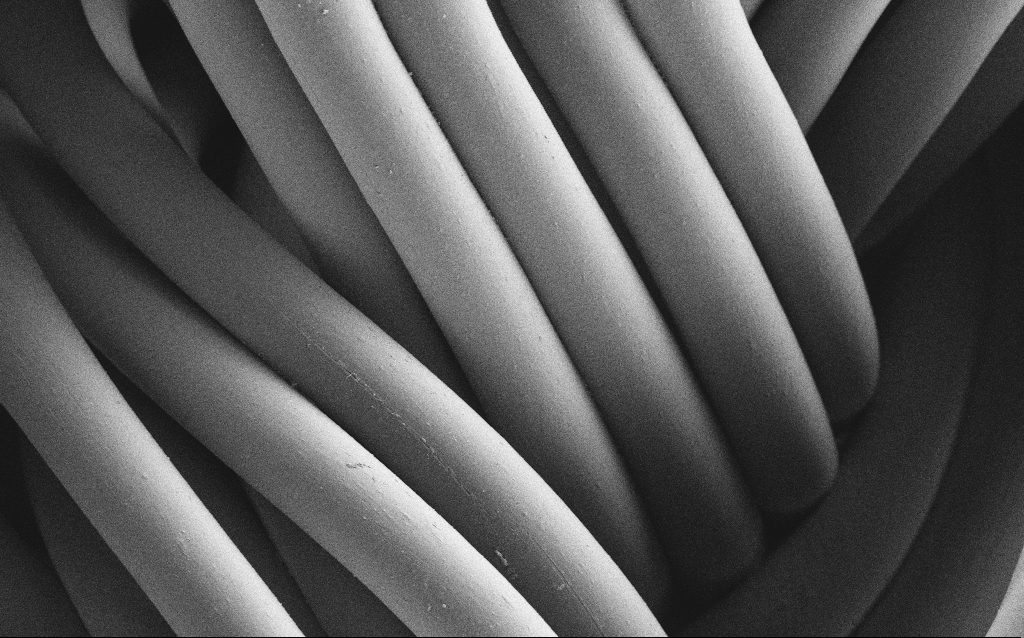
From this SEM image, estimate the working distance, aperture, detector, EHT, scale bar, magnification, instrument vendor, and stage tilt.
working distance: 4 mm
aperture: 30 µm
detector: SE2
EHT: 1 kV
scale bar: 20000 nm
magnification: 1.6 K X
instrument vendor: Zeiss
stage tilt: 0°